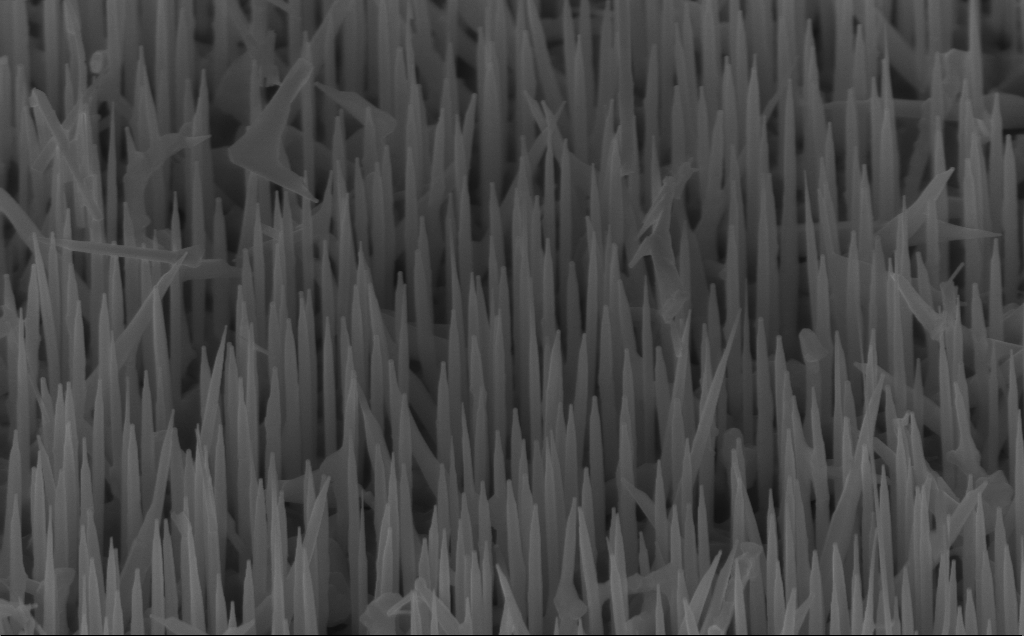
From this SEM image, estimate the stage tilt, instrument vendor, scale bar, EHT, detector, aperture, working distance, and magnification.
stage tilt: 45°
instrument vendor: Zeiss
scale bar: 1000 nm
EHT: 10 kV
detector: InLens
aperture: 30 µm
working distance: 7 mm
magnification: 40 K X